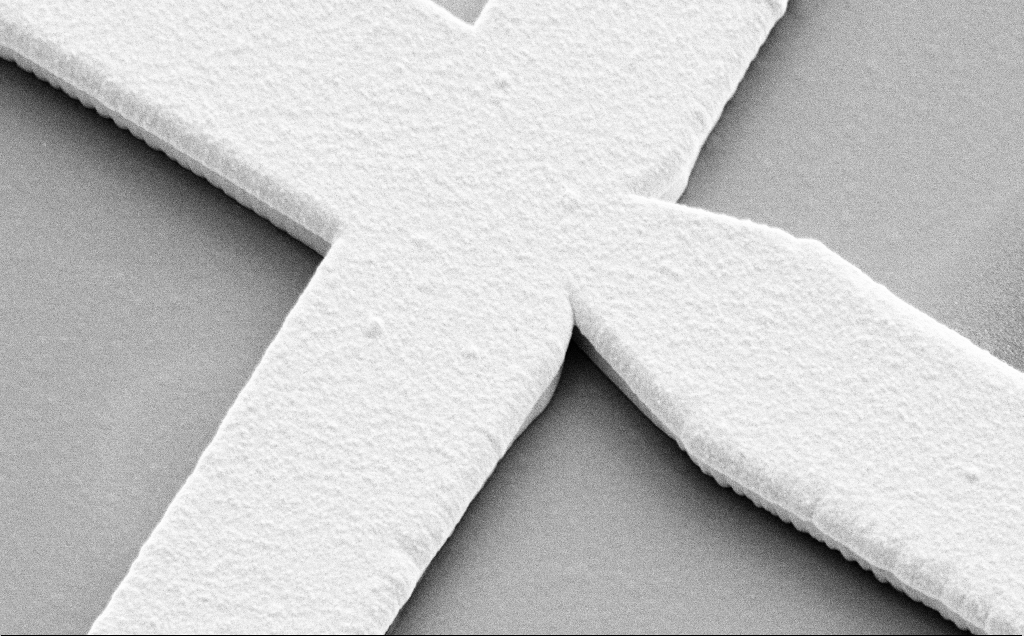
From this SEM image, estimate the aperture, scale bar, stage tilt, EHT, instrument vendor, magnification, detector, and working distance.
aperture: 30 µm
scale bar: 2000 nm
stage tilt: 45°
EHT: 10 kV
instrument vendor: Zeiss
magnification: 8.05 K X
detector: SE2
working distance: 12 mm